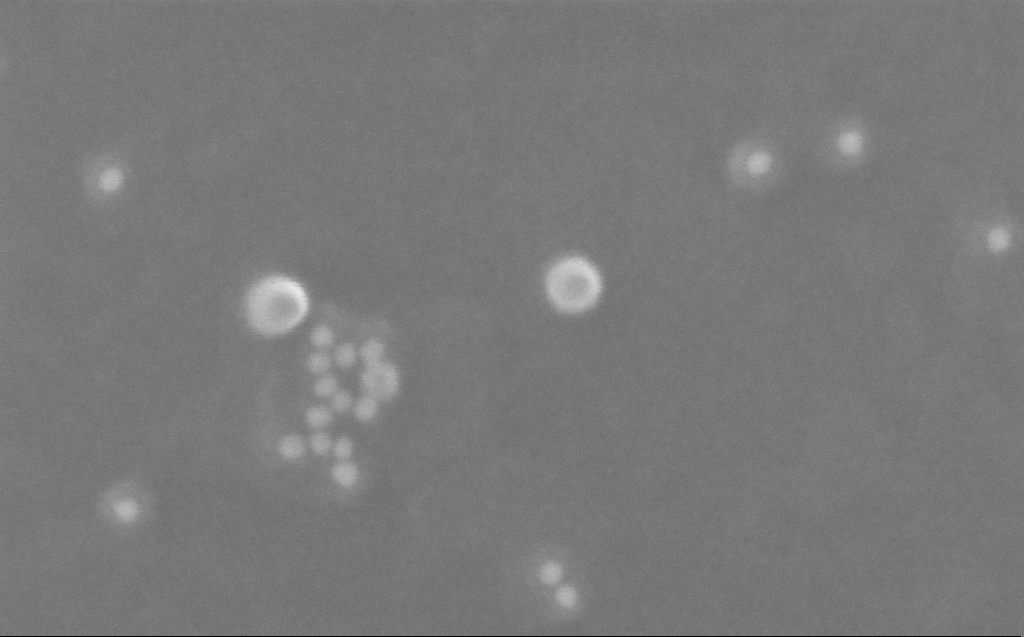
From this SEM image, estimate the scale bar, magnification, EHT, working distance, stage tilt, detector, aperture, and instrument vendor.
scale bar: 100 nm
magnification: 562.1 K X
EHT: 10 kV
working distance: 3 mm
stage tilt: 0°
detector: InLens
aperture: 30 µm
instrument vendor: Zeiss